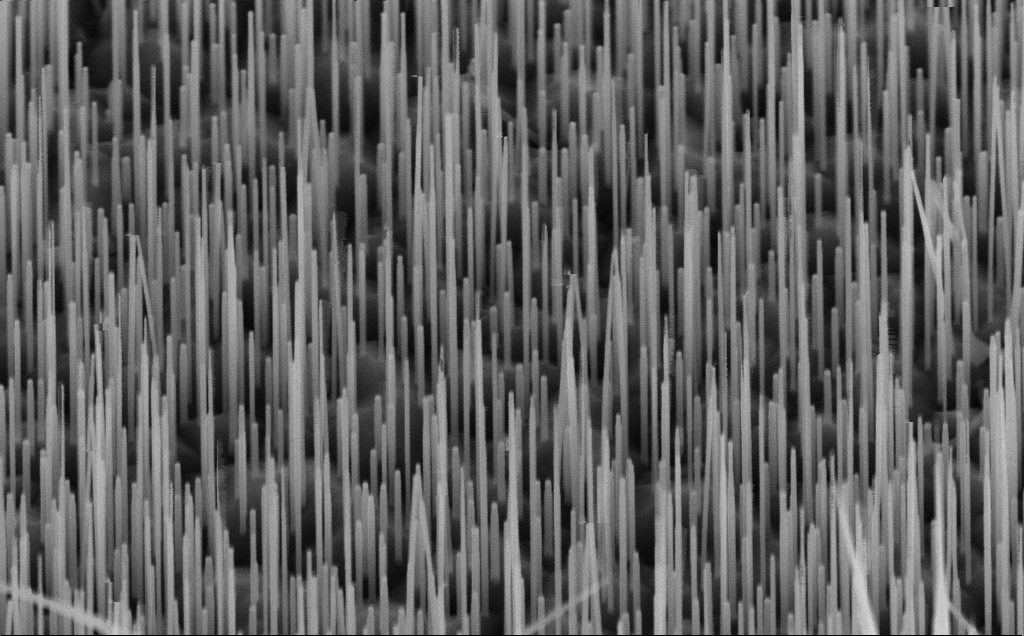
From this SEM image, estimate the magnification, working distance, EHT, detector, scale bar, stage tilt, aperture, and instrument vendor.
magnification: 60 K X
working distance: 7 mm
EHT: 10 kV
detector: InLens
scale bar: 1000 nm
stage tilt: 45°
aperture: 30 µm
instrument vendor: Zeiss